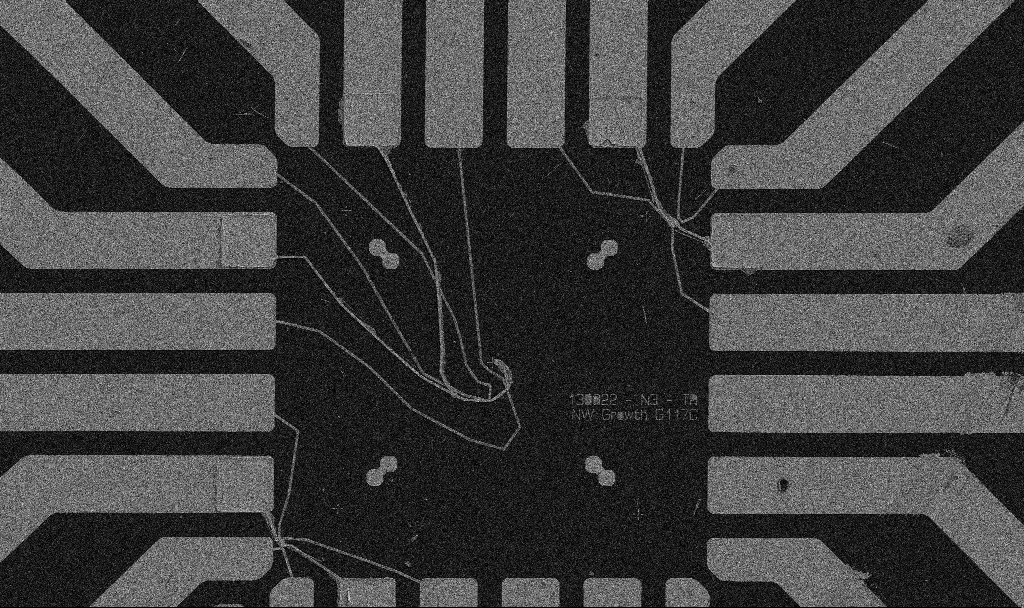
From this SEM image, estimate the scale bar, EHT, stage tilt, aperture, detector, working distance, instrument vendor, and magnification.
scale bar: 20000 nm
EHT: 5 kV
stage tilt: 0°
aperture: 30 µm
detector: SE2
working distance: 10.7 mm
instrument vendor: Zeiss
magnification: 1 K X